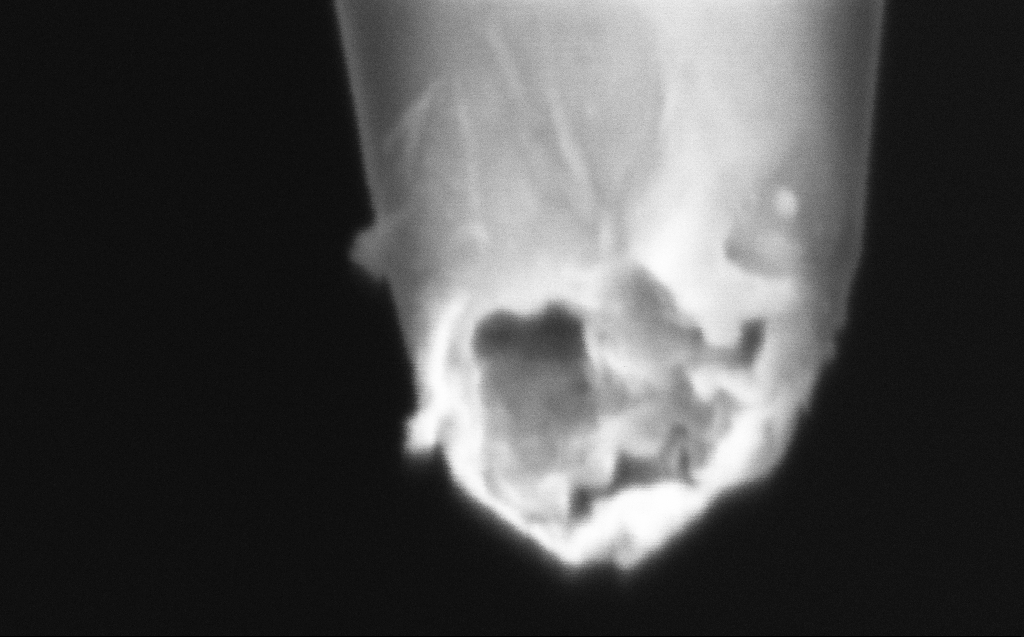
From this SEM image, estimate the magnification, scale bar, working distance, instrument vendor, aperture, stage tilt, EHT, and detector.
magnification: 500 K X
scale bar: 100 nm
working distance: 6 mm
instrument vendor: Zeiss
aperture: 30 µm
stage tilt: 45°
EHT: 2 kV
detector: InLens